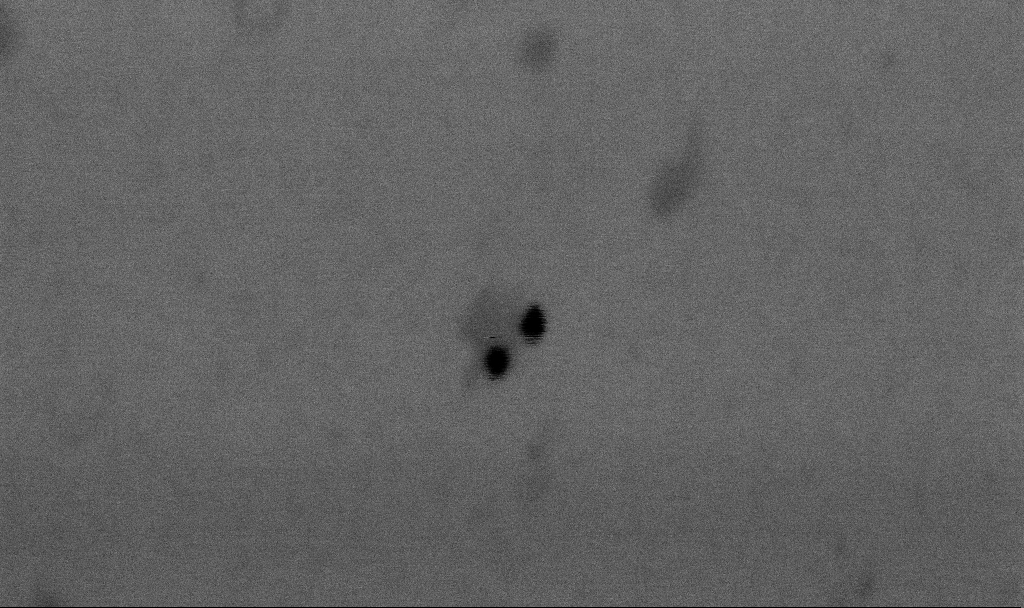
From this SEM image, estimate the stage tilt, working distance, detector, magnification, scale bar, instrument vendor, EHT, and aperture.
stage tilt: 0°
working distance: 6.6 mm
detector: SE2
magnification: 350 K X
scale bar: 100 nm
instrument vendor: Zeiss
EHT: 2 kV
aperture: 30 µm